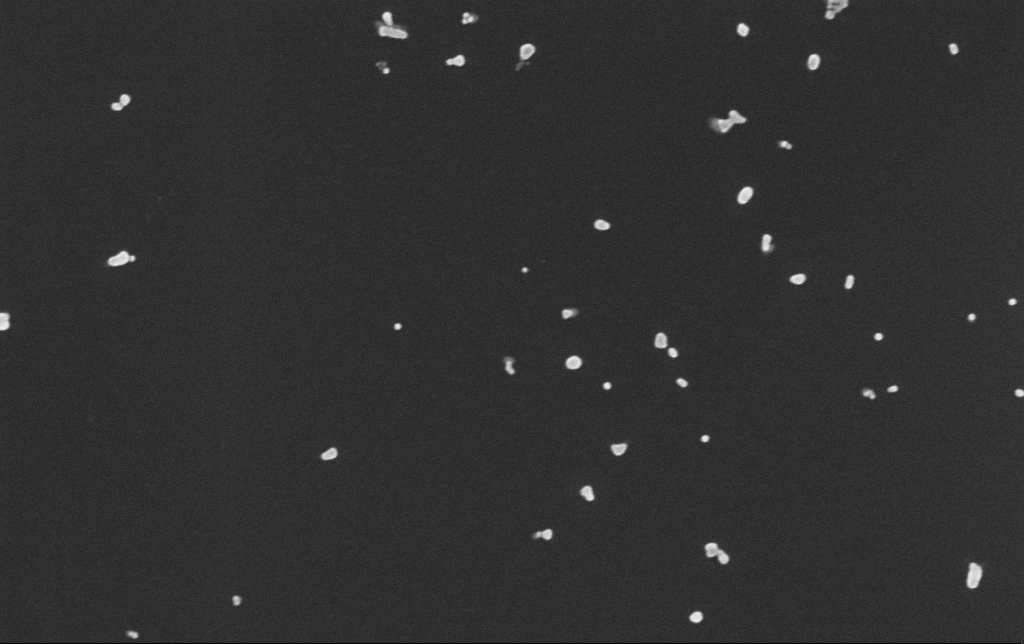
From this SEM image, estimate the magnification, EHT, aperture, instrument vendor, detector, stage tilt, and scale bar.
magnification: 100 K X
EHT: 10 kV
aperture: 30 µm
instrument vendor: Zeiss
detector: InLens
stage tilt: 0°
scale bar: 200 nm